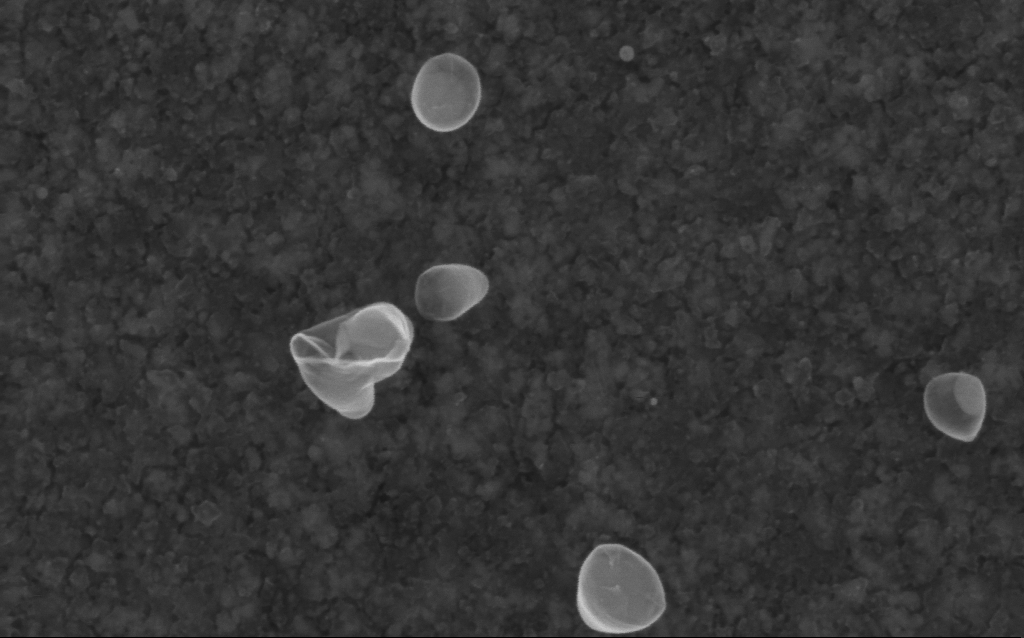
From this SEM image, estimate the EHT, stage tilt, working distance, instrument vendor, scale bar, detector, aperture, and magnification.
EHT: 5 kV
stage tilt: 0°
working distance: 2.6 mm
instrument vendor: Zeiss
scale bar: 100 nm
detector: InLens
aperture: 30 µm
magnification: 260.22 K X